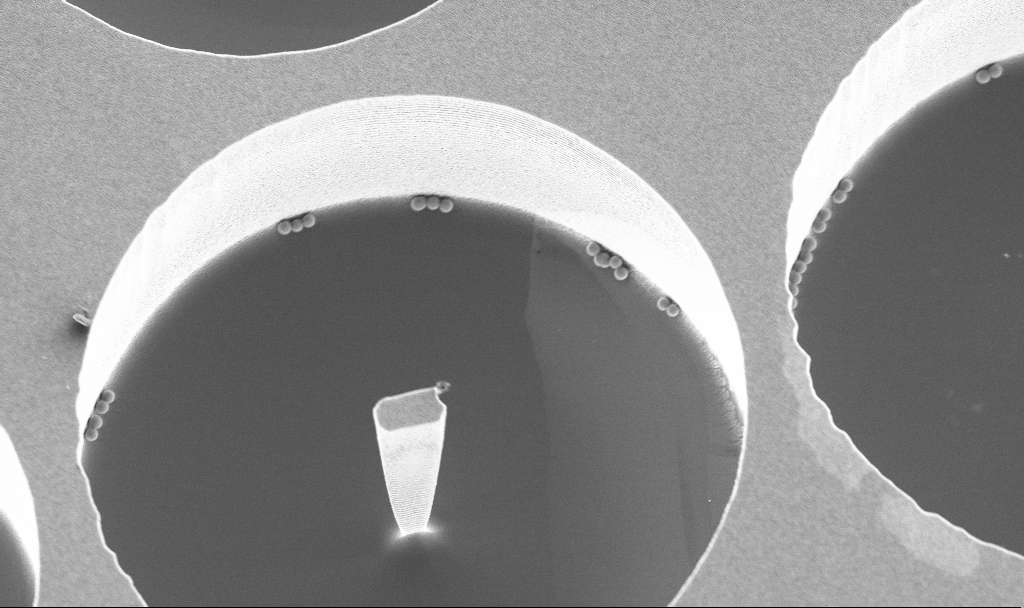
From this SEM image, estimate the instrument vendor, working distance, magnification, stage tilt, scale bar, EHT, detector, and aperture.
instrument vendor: Zeiss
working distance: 3.8 mm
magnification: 8.1 K X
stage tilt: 20°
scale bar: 2000 nm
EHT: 3 kV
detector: InLens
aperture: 30 µm